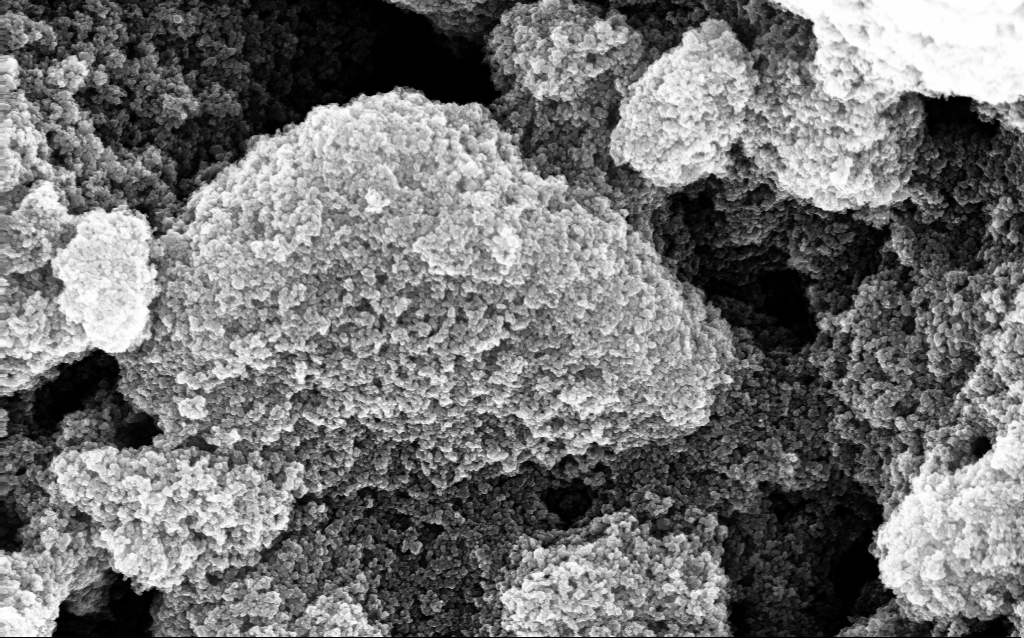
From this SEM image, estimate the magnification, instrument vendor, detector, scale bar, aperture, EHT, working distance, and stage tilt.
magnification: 71.61 K X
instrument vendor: Zeiss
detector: InLens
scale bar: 1000 nm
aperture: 30 µm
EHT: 10 kV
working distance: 1.8 mm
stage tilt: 0°